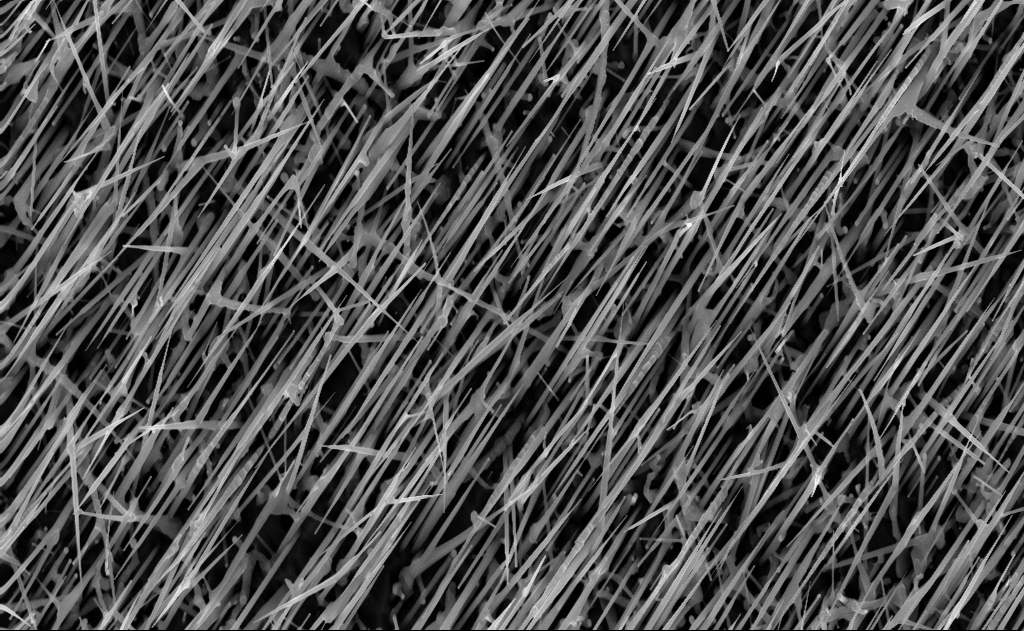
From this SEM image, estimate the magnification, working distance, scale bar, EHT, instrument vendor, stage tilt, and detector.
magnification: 20 K X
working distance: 7 mm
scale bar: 2000 nm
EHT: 10 kV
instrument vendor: Zeiss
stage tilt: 0°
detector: InLens